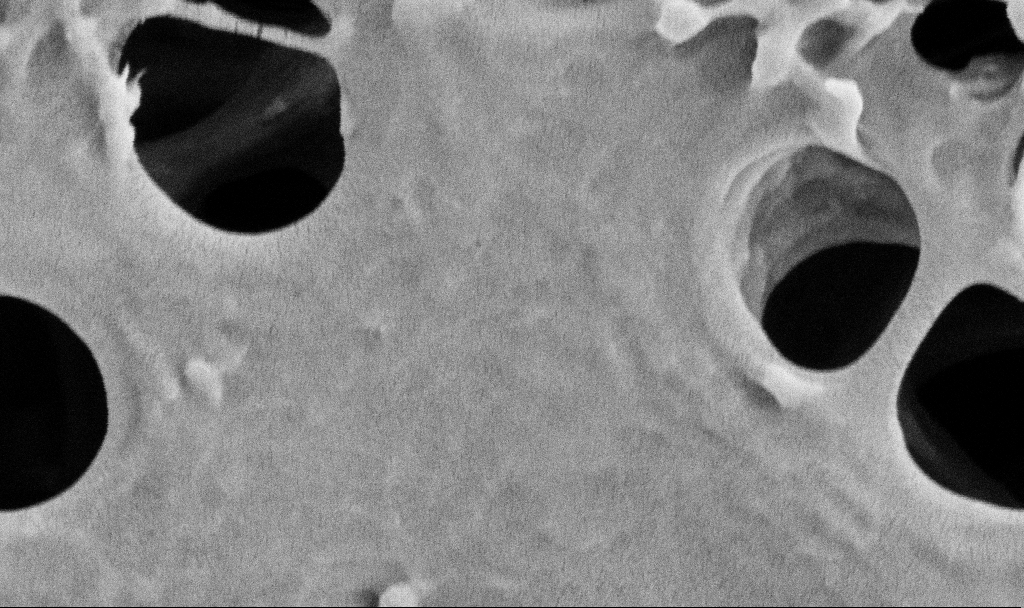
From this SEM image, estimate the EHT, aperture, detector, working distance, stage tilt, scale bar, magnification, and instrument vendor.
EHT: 2 kV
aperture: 30 µm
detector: SE2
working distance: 3.7 mm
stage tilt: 0°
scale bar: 200 nm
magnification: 100 K X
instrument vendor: Zeiss